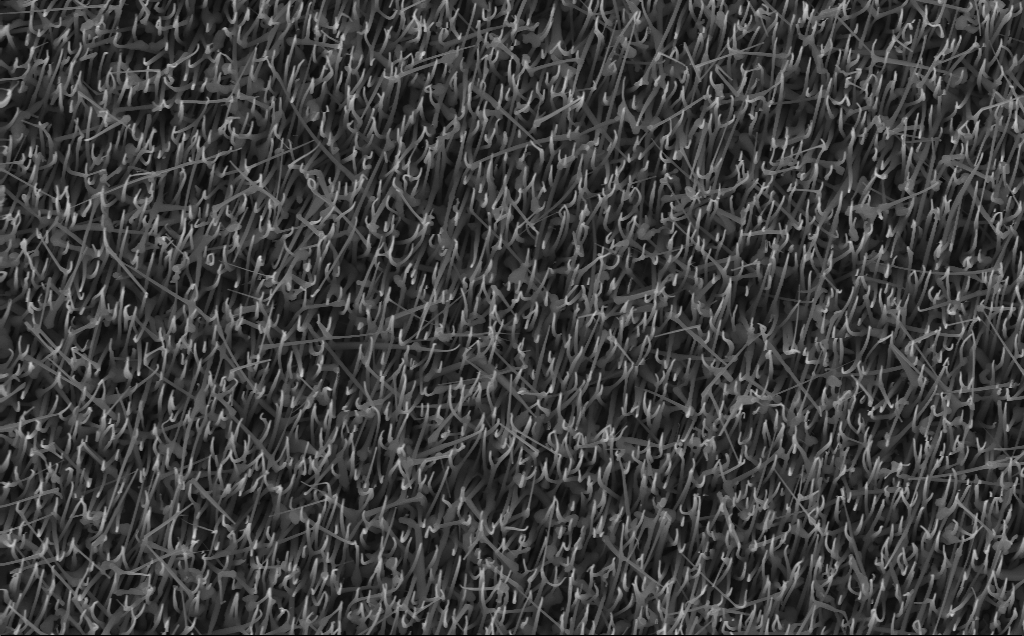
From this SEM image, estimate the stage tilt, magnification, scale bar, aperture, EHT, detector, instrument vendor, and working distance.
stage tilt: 0°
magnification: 20 K X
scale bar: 2000 nm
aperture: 30 µm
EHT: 10 kV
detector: InLens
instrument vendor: Zeiss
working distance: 4 mm